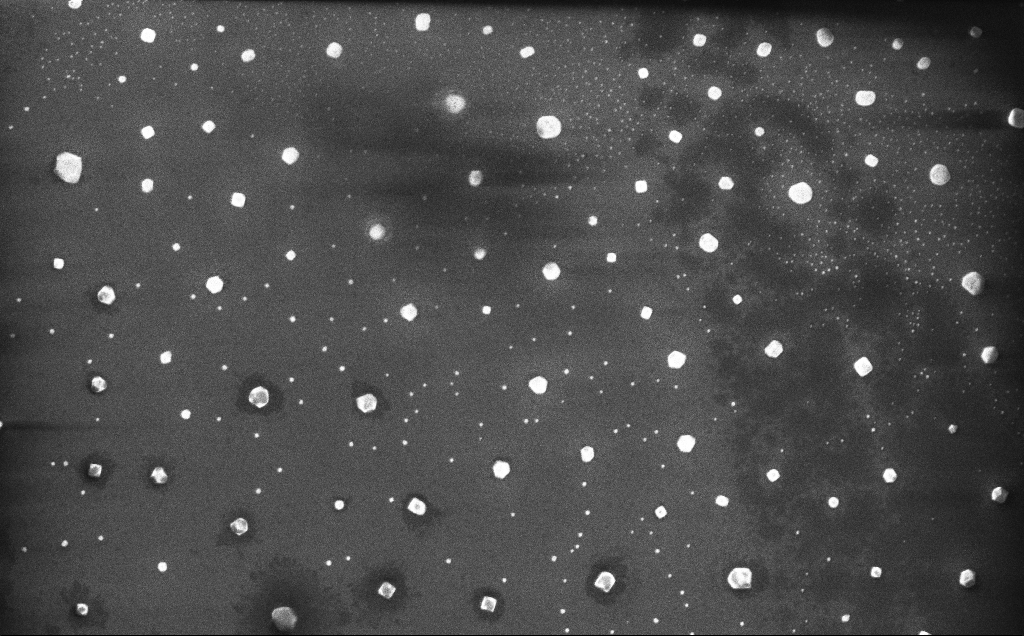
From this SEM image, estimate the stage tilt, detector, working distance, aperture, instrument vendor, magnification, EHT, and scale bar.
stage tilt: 0°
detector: InLens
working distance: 5 mm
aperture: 30 µm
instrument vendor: Zeiss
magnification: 21.66 K X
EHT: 10 kV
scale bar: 1000 nm